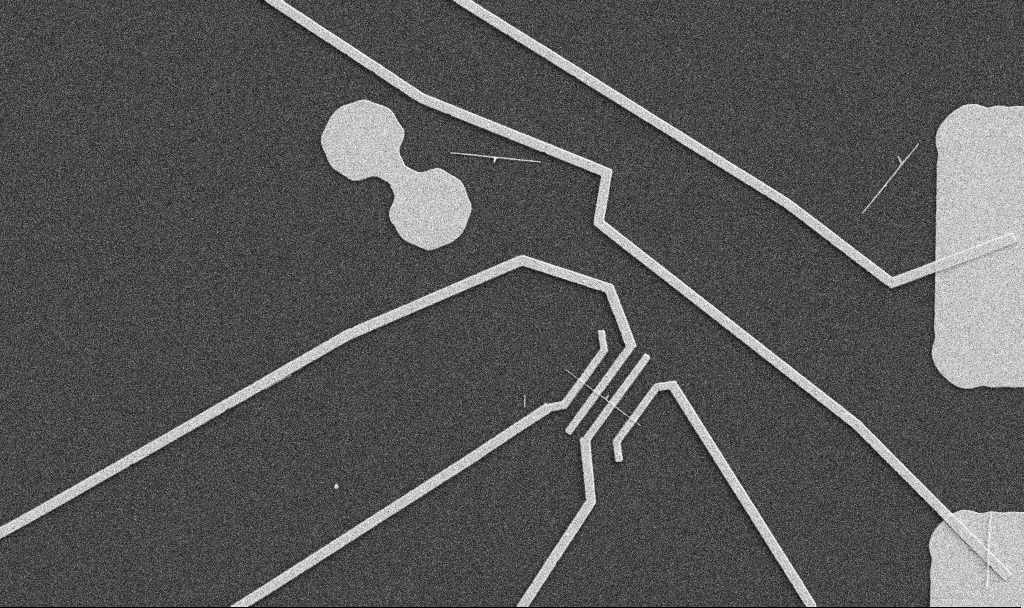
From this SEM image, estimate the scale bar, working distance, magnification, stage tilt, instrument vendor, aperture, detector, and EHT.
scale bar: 10000 nm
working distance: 10.7 mm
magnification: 5 K X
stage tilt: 0°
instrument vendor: Zeiss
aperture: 30 µm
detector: SE2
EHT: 5 kV